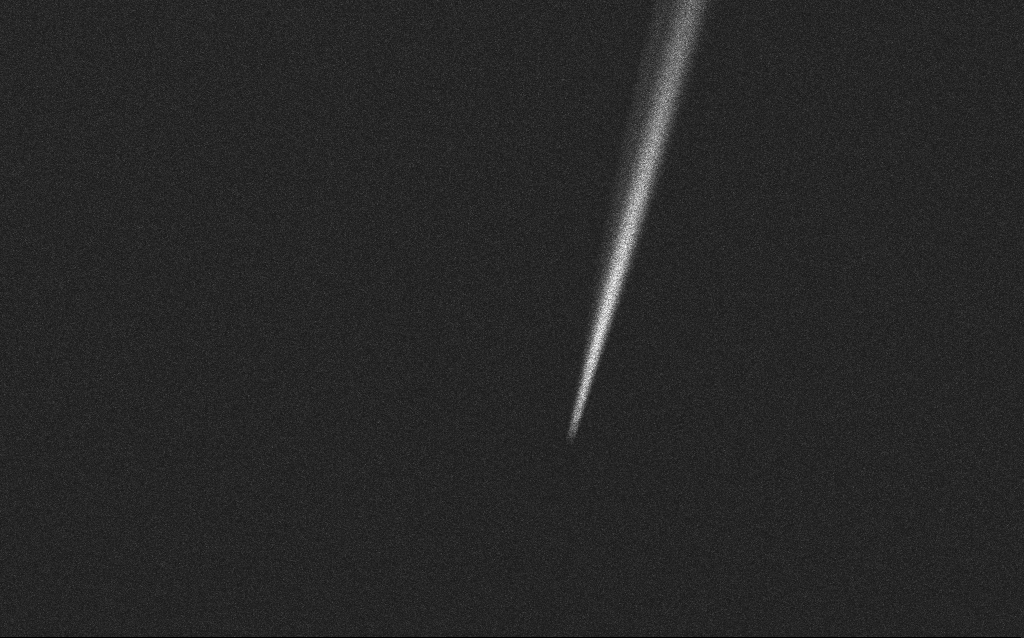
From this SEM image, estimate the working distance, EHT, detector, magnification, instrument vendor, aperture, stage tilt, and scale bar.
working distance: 5 mm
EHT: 2.5 kV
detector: InLens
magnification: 1 K X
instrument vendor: Zeiss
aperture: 30 µm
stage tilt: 45°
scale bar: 20000 nm